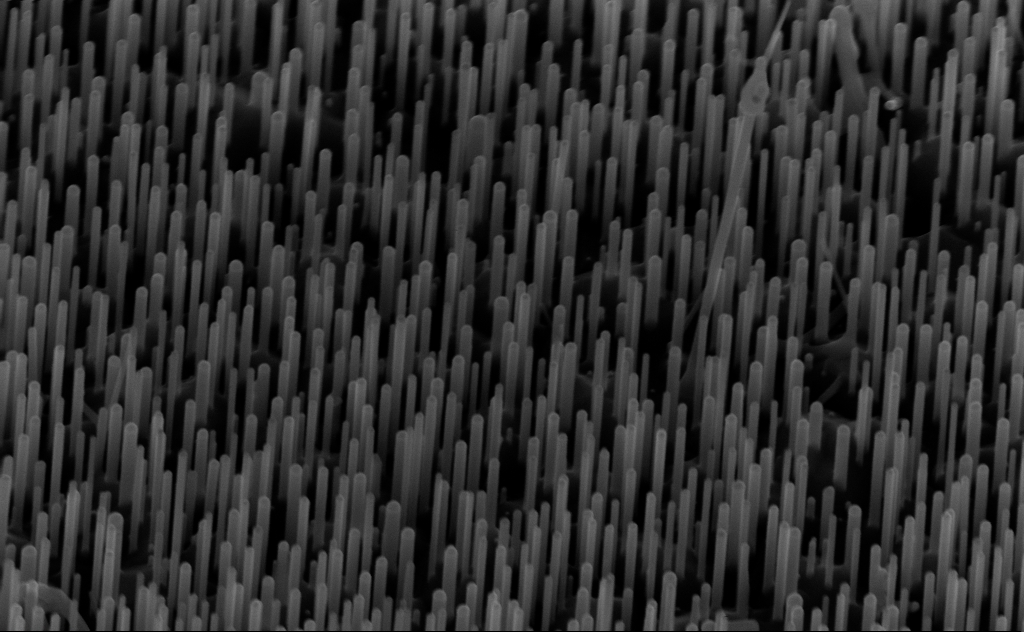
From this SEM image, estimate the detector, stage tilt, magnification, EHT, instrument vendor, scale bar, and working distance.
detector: InLens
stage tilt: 45°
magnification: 80 K X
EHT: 10 kV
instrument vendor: Zeiss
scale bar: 200 nm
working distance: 6 mm